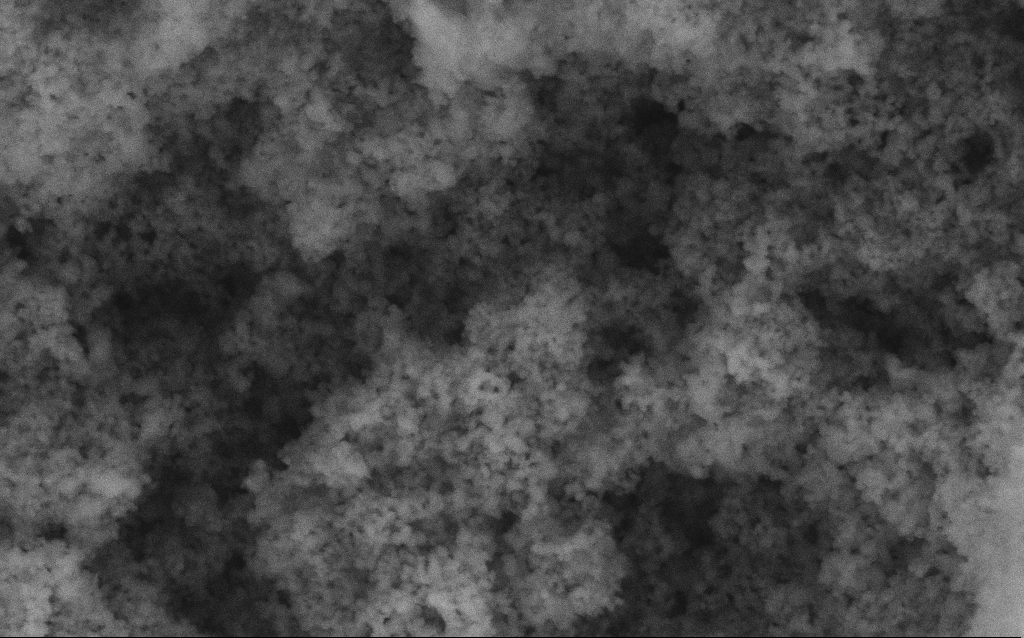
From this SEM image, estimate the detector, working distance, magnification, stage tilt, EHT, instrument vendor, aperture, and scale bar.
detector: SE2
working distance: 4.2 mm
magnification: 114.64 K X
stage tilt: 0°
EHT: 5 kV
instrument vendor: Zeiss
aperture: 30 µm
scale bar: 200 nm